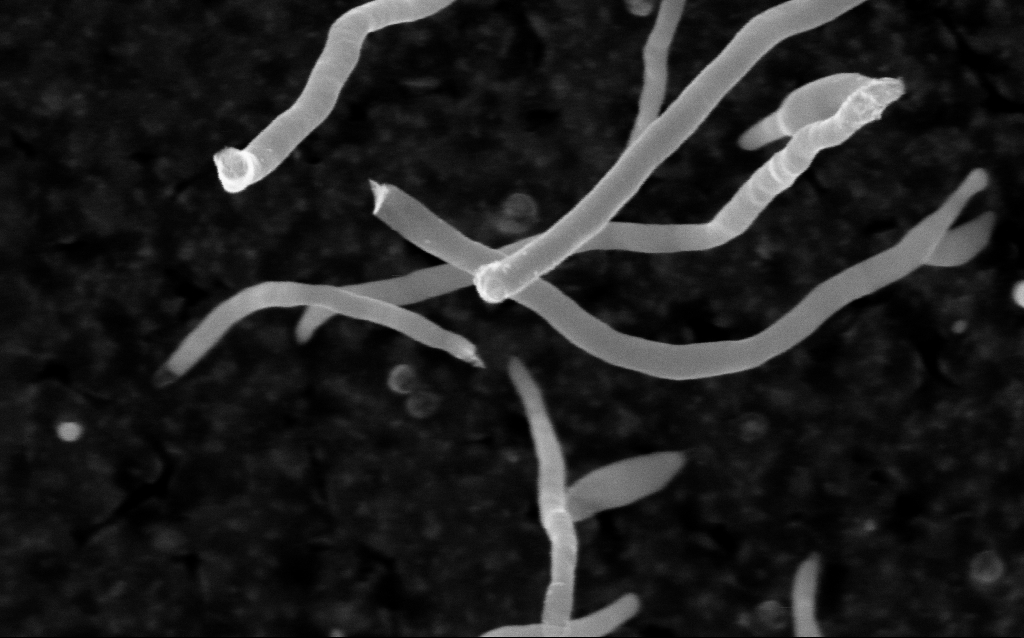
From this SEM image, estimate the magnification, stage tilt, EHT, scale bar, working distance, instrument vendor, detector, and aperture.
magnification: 200 K X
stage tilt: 0°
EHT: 5 kV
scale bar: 200 nm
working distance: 1.8 mm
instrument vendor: Zeiss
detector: InLens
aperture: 30 µm